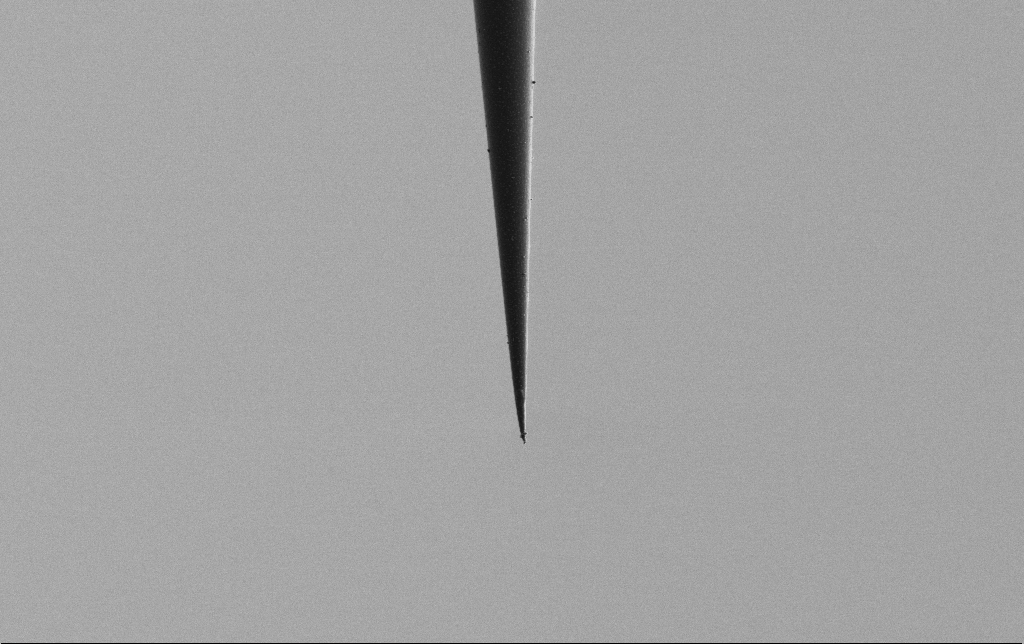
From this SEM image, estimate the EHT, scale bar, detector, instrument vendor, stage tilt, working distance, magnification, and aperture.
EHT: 2 kV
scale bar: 10000 nm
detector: SE2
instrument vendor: Zeiss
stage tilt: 0°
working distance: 6.5 mm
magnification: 5 K X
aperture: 30 µm